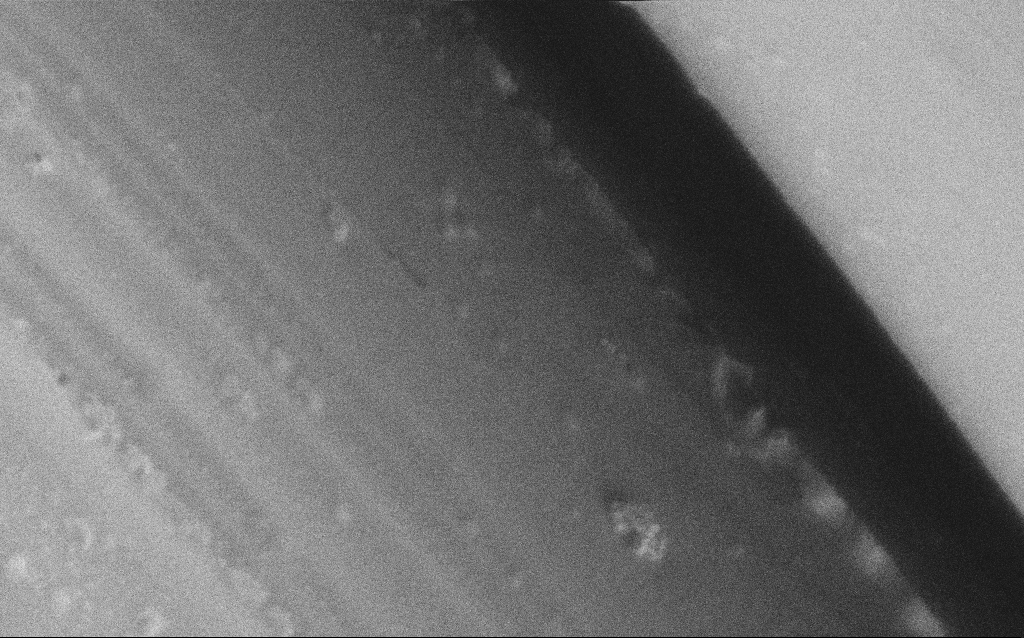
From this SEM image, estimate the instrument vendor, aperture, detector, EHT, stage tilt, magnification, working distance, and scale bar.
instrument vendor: Zeiss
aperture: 30 µm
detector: SE2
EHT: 1 kV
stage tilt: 0°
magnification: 39.36 K X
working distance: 4 mm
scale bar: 1000 nm